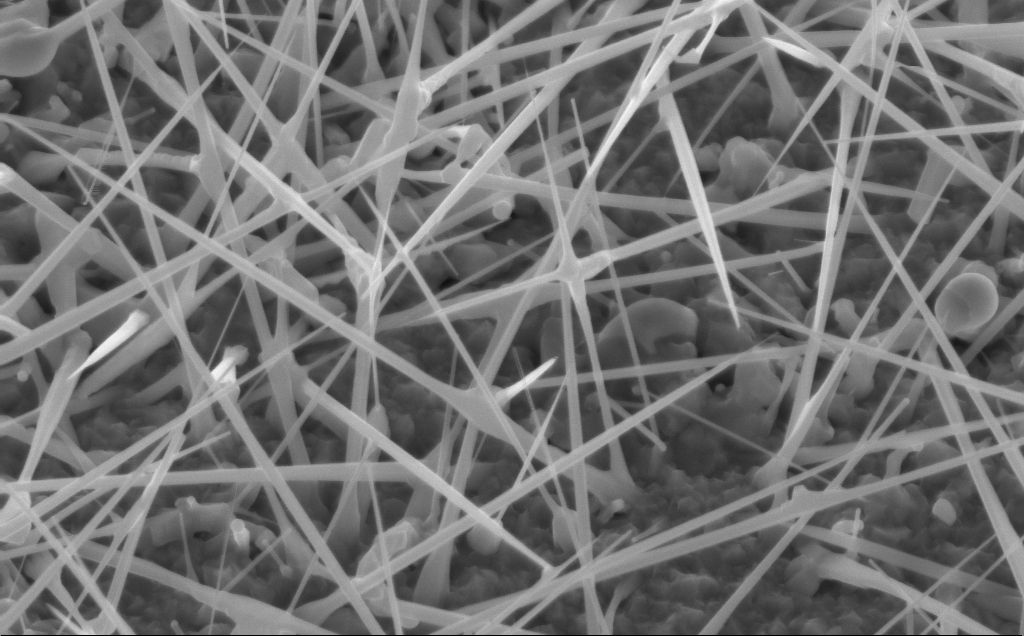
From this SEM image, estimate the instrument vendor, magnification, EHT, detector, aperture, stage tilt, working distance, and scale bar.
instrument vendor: Zeiss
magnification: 40 K X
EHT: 10 kV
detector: InLens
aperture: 30 µm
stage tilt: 30°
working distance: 5 mm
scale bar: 1000 nm